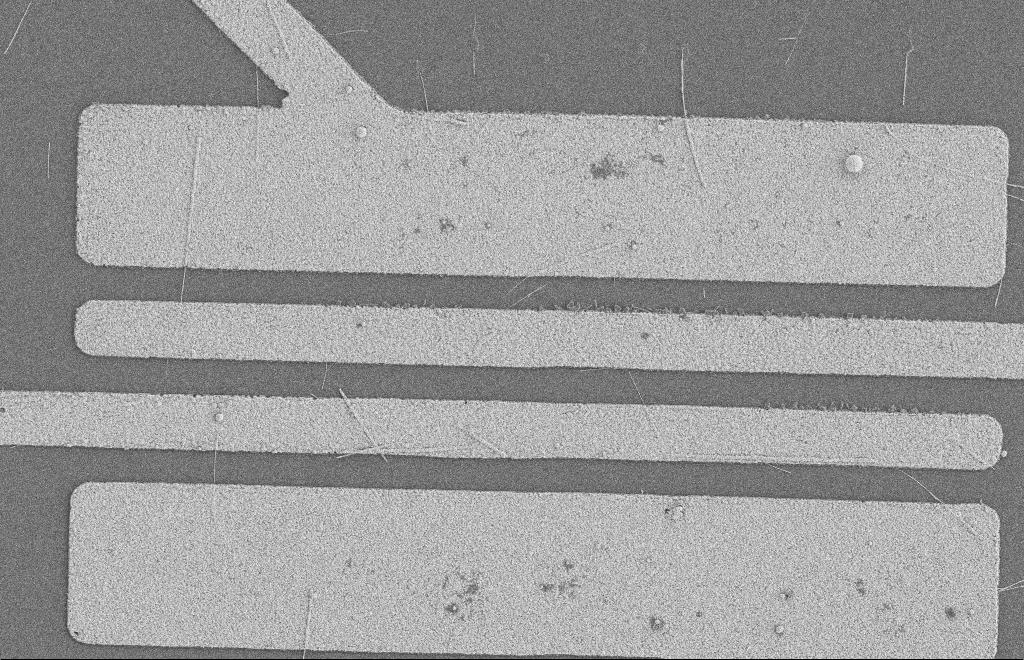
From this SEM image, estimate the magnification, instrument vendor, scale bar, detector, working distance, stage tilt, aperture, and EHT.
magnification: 5.58 K X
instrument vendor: Zeiss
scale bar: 2000 nm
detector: SE2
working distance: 8 mm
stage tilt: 0°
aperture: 20 µm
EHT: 2 kV